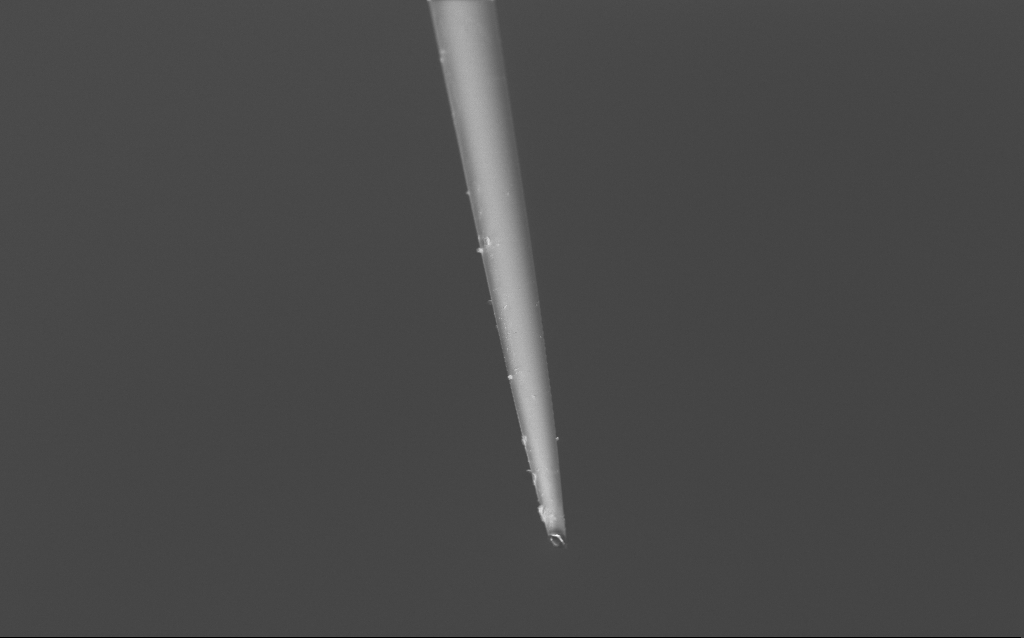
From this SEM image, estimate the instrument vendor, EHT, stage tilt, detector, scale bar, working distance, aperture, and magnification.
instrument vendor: Zeiss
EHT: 2 kV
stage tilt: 45°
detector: InLens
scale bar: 20000 nm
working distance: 6 mm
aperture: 30 µm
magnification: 1 K X